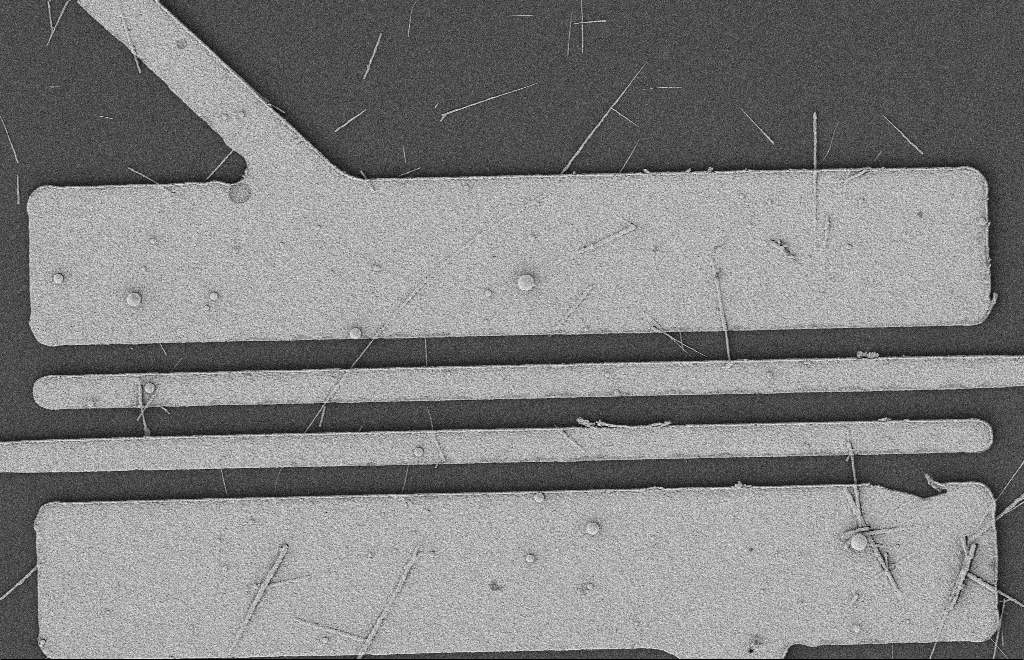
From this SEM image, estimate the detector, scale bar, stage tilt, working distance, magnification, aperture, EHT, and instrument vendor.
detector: SE2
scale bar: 2000 nm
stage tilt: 0°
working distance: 12 mm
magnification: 5.75 K X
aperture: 20 µm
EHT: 2 kV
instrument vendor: Zeiss